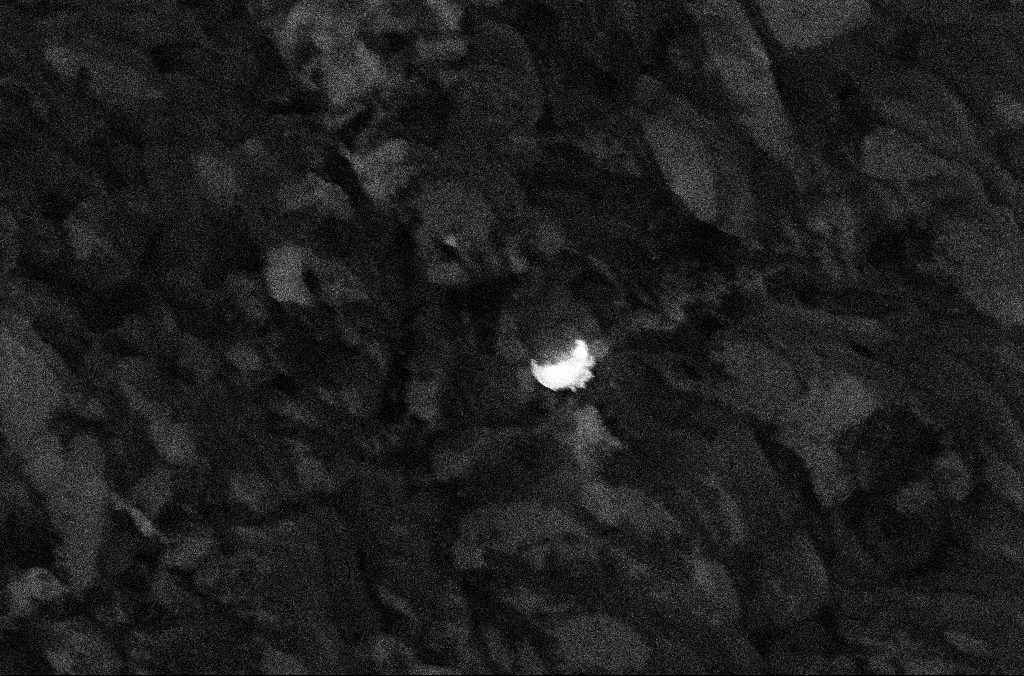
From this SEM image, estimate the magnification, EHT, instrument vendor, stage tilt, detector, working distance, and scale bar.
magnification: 50 K X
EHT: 5 kV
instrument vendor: Zeiss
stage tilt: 0°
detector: InLens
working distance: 6.7 mm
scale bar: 1000 nm